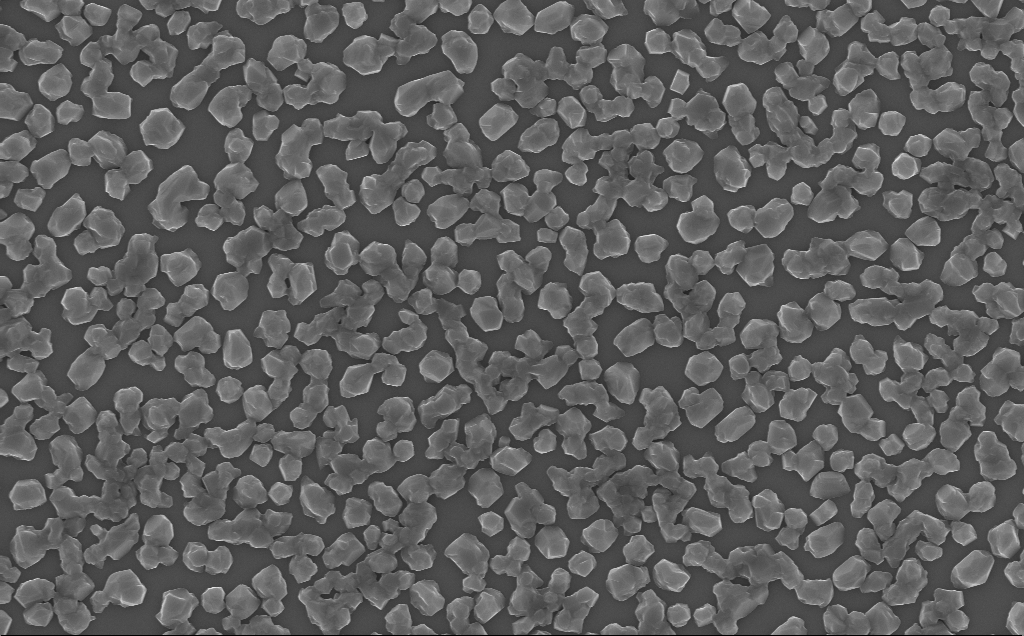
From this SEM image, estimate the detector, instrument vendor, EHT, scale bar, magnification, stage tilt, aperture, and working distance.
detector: InLens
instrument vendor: Zeiss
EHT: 10 kV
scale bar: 1000 nm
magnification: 20 K X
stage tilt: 0°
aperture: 30 µm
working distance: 6 mm